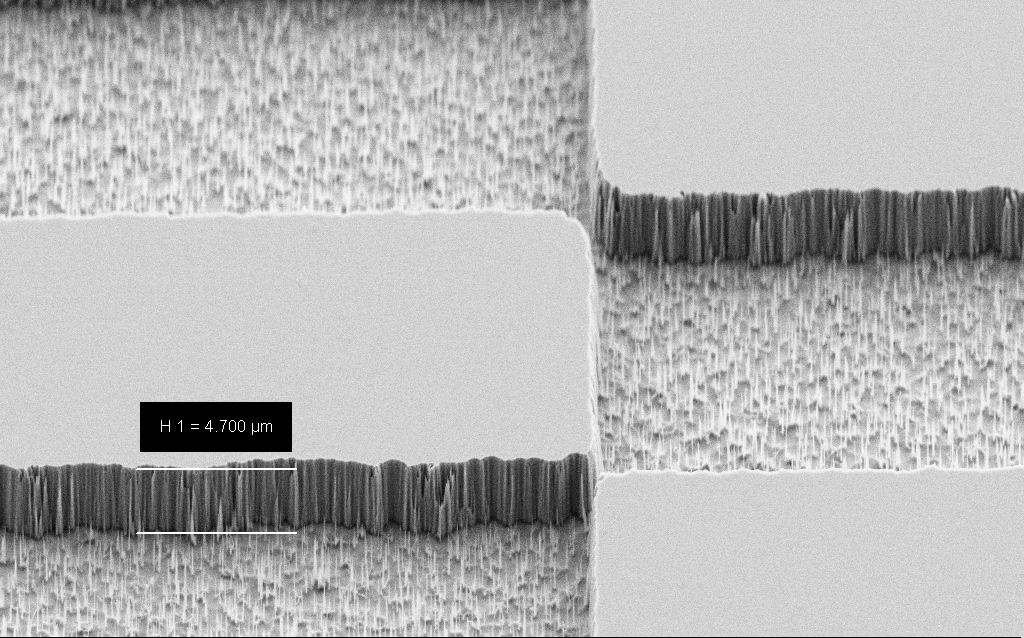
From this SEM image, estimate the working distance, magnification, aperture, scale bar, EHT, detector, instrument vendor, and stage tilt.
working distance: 7 mm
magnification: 5 K X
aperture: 30 µm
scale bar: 10000 nm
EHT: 5 kV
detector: SE2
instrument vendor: Zeiss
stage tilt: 45°